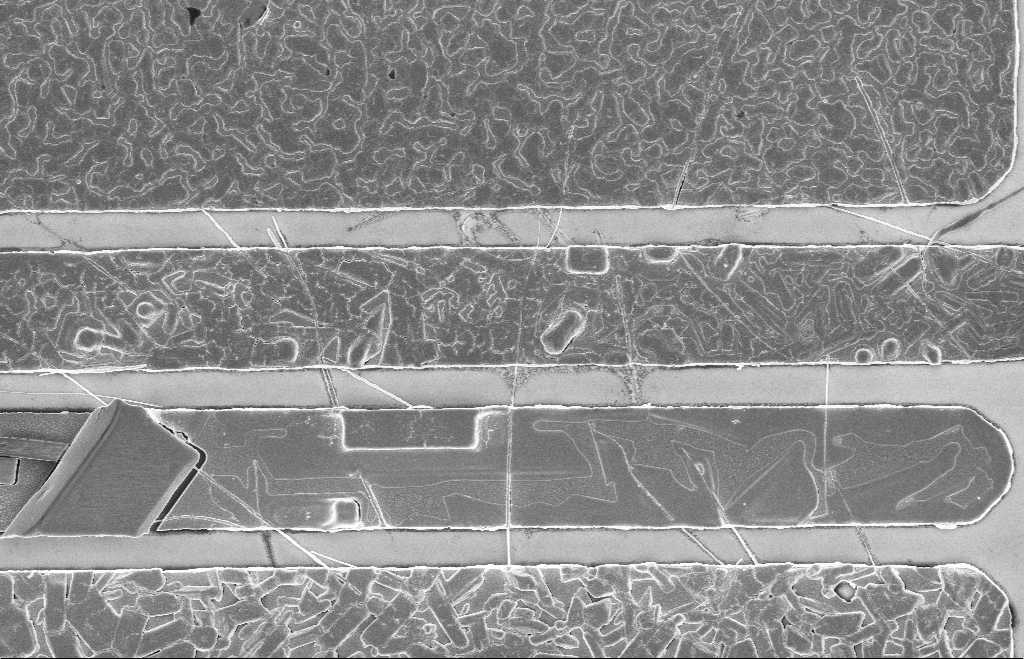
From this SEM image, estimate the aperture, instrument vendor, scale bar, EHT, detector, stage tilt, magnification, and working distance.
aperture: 20 µm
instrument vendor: Zeiss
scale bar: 2000 nm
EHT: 5 kV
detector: InLens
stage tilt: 0°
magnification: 9.88 K X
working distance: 8 mm